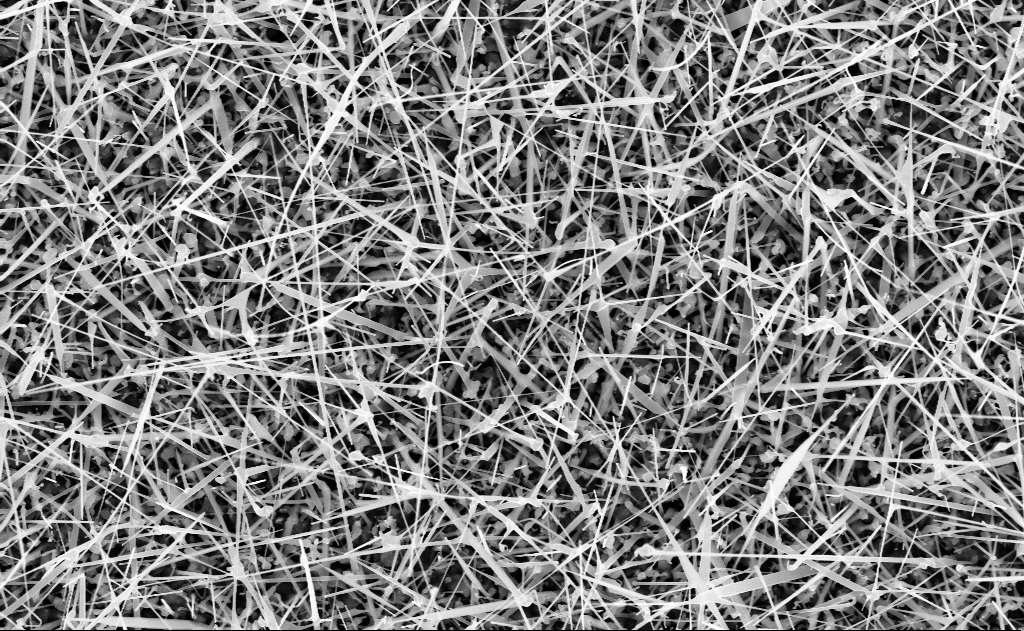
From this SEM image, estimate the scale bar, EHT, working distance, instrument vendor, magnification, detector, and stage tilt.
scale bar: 1000 nm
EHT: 10 kV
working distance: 11 mm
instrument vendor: Zeiss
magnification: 20 K X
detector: InLens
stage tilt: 0°